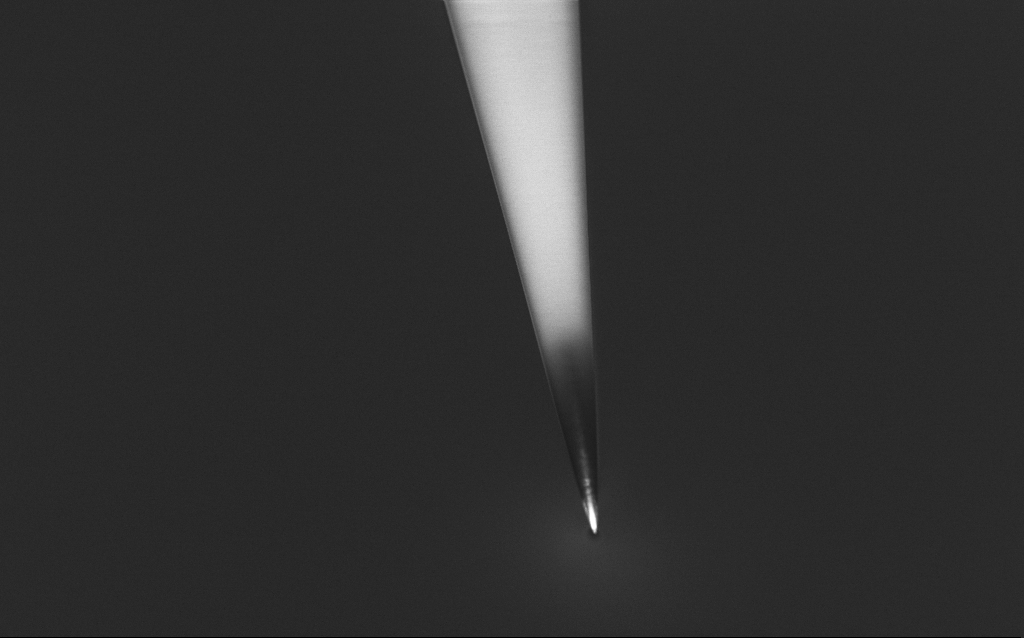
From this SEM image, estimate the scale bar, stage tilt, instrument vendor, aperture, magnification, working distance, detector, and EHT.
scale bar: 10000 nm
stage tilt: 45°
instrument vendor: Zeiss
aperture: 30 µm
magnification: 5 K X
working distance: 6 mm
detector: InLens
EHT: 1 kV